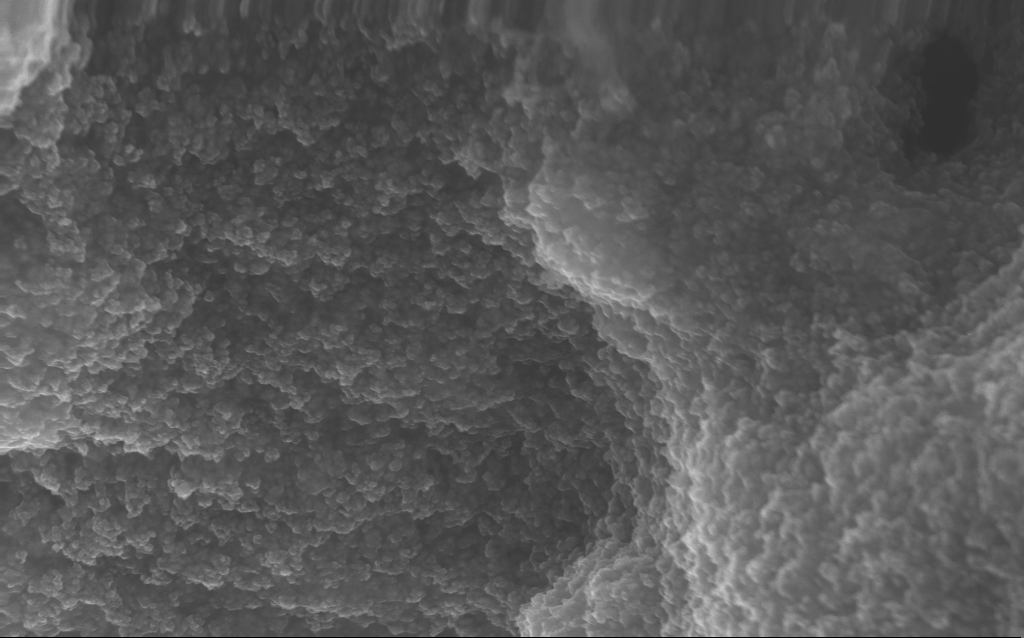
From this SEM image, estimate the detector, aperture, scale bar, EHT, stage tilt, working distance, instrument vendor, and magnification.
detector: InLens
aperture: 30 µm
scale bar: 1000 nm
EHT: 10 kV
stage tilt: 0°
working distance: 2.8 mm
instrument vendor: Zeiss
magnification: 65.04 K X